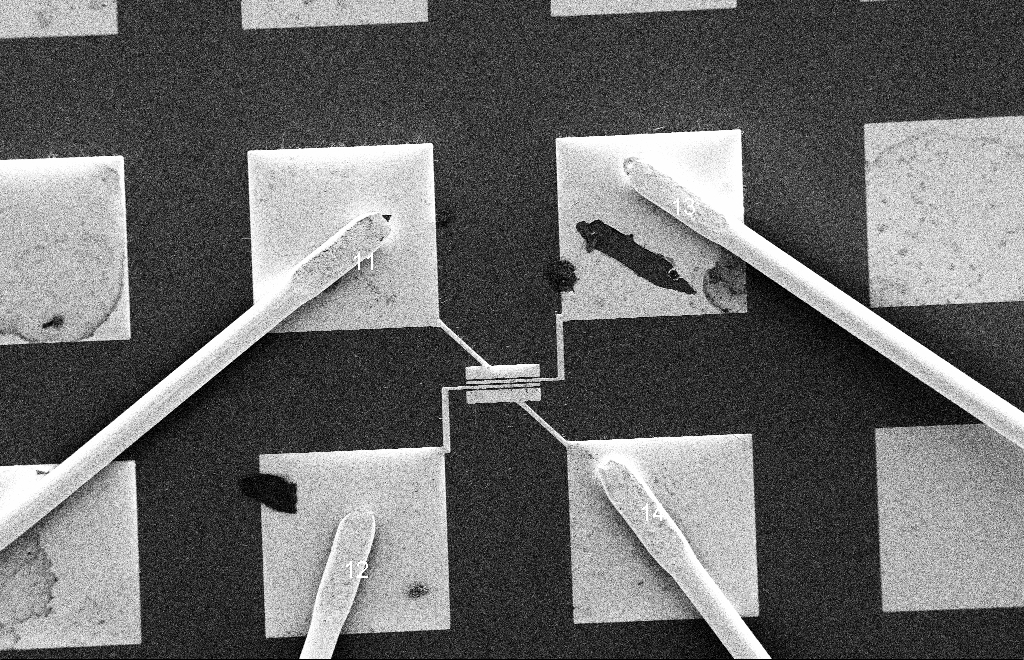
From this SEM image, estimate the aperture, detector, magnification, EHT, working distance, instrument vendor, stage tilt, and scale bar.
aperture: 20 µm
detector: SE2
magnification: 0.446 K X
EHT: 2 kV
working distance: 9 mm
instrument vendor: Zeiss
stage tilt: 0°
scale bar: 100000 nm